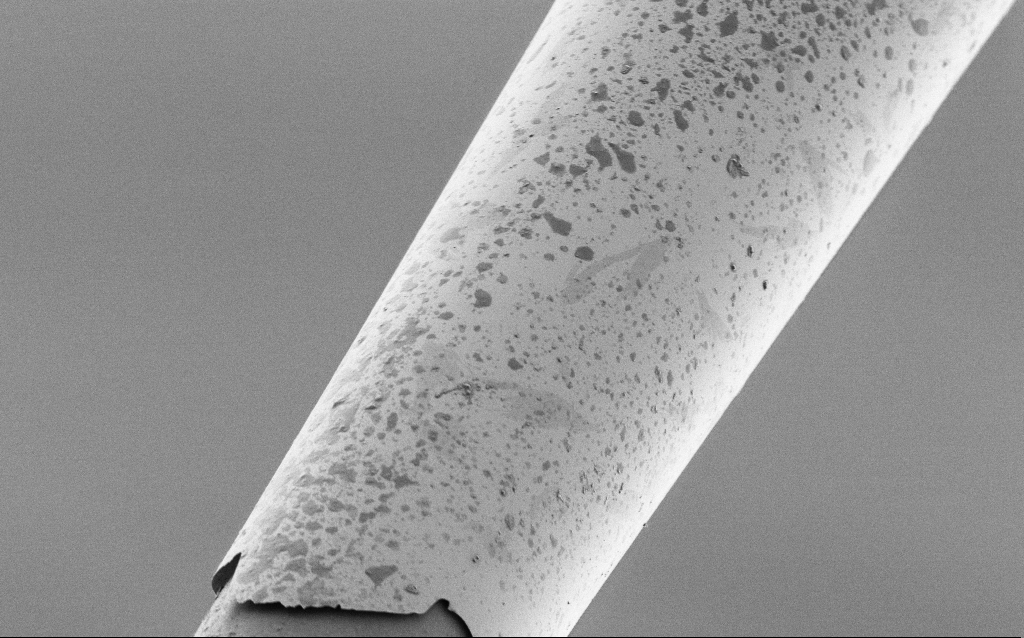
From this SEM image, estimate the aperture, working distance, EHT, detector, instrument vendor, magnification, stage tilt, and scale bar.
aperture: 30 µm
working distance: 7.7 mm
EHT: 1 kV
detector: SE2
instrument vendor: Zeiss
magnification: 2.5 K X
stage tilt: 45°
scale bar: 20000 nm